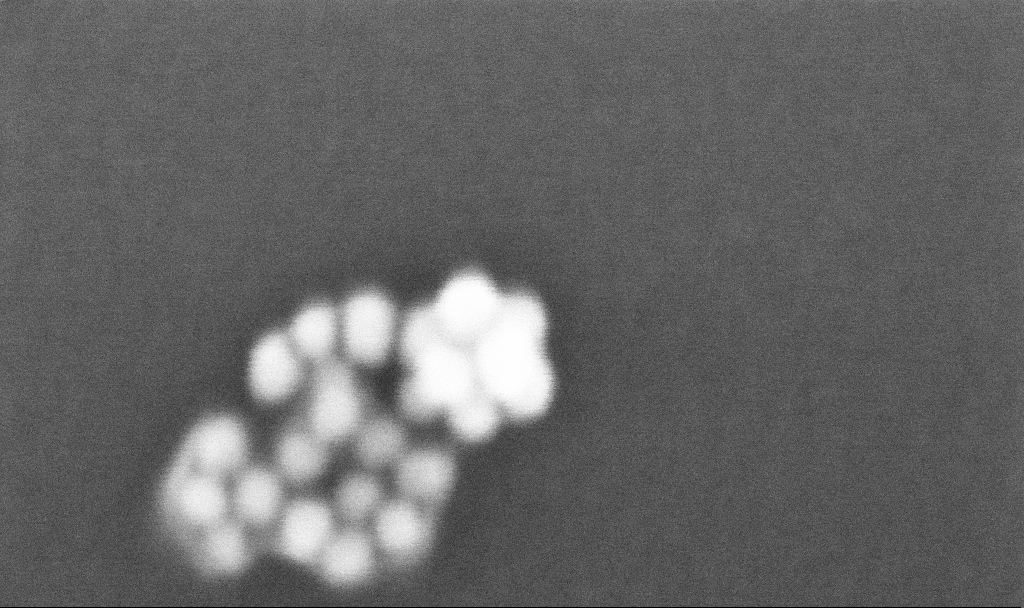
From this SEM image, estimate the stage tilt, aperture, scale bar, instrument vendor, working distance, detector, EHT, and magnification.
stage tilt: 0°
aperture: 30 µm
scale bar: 20 nm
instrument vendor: Zeiss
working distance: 3.4 mm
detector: InLens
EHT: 10 kV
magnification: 891.23 K X